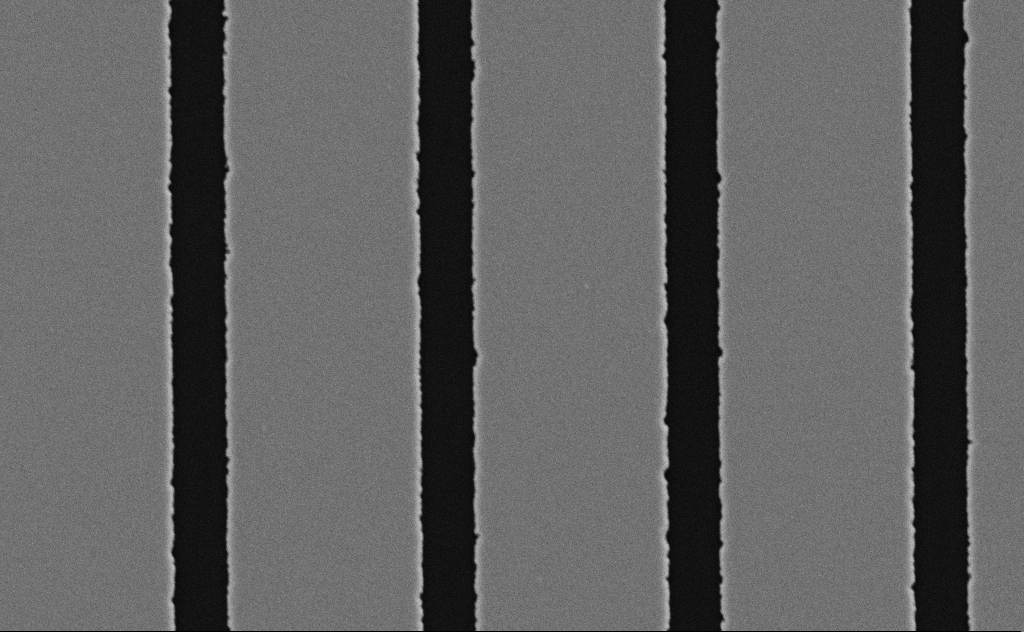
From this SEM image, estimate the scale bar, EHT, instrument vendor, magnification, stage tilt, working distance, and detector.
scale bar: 2000 nm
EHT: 5 kV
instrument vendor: Zeiss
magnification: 22.55 K X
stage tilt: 0°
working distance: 8 mm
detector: SE2